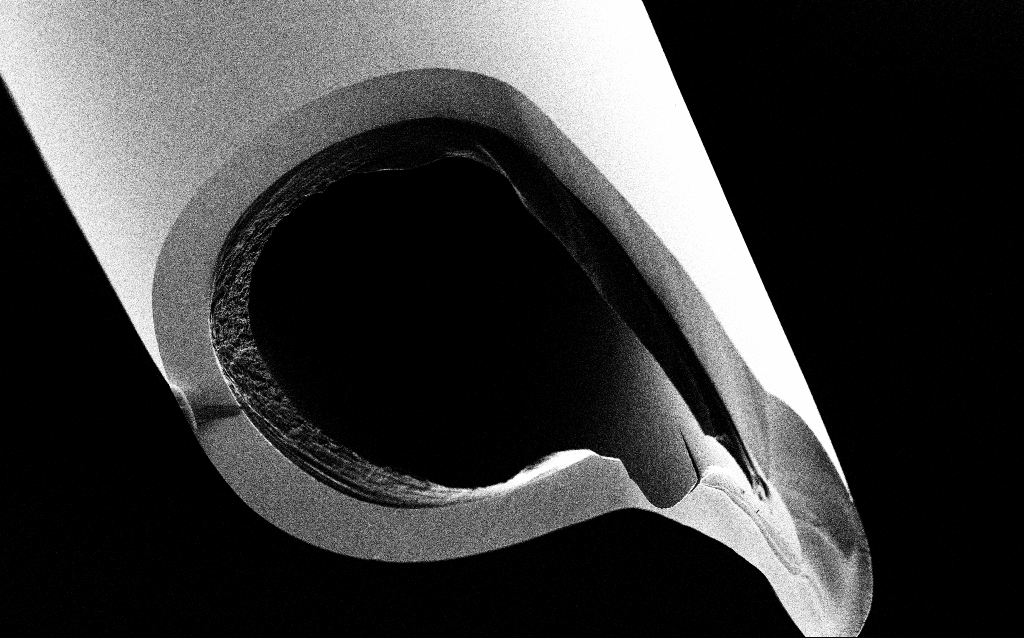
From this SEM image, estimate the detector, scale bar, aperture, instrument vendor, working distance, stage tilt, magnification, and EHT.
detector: SE2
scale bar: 10000 nm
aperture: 30 µm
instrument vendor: Zeiss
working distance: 6.4 mm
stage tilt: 45°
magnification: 5 K X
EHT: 1 kV